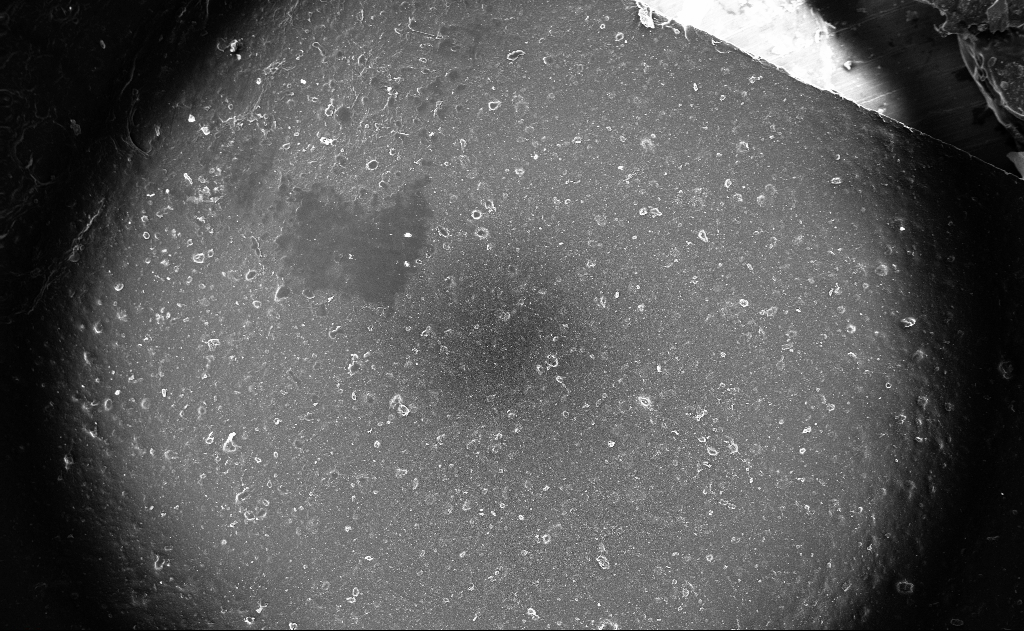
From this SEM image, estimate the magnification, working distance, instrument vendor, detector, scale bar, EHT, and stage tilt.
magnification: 0.122 K X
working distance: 3 mm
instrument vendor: Zeiss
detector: InLens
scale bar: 100000 nm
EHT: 10 kV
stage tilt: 0°